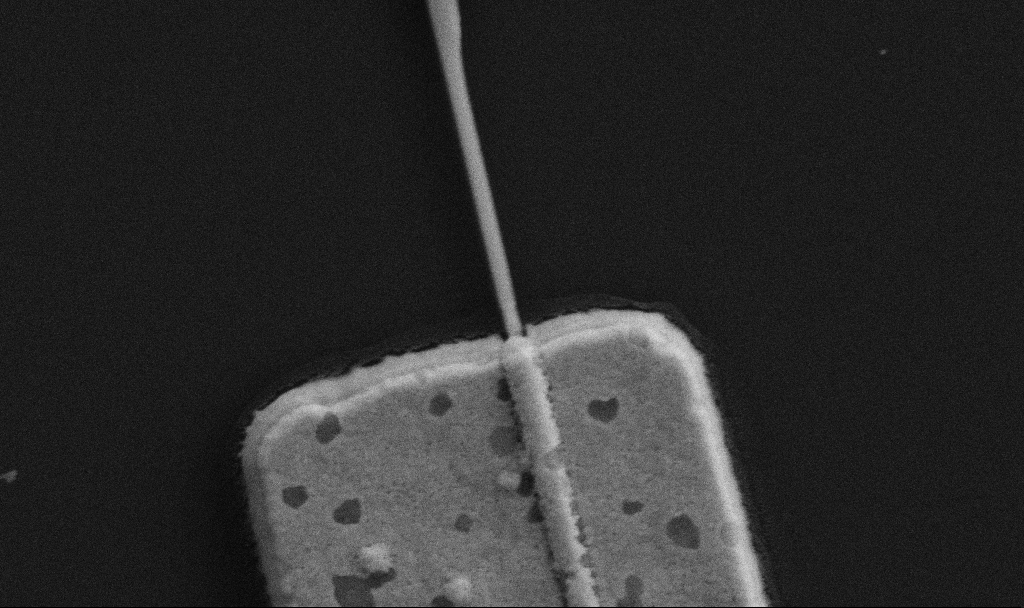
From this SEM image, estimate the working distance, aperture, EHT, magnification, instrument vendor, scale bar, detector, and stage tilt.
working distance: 8.7 mm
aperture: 30 µm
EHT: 5 kV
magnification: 80 K X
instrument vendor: Zeiss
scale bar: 200 nm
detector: SE2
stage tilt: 0°